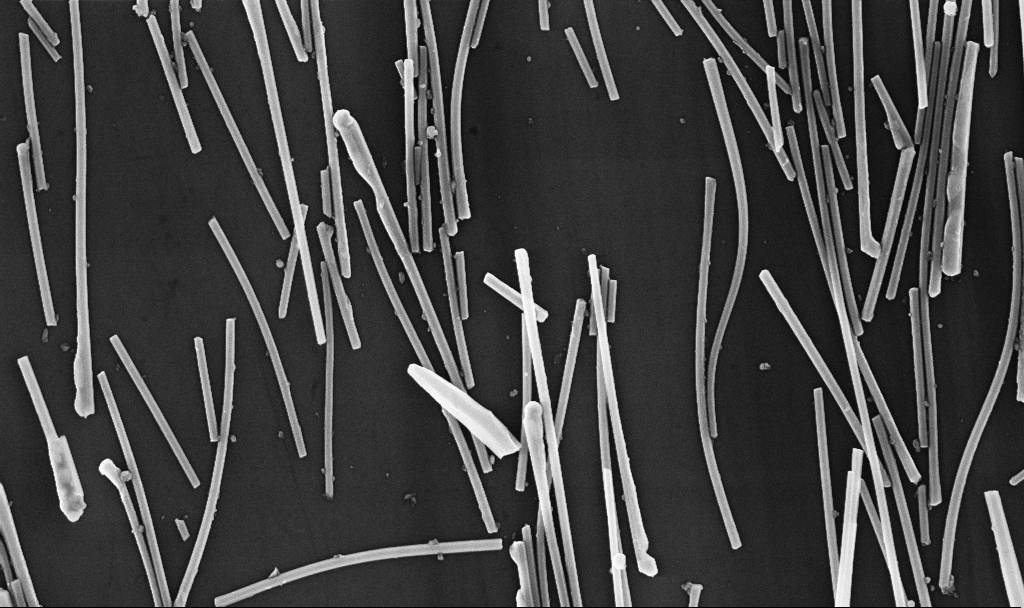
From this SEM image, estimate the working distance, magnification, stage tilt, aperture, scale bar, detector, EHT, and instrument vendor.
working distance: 6.7 mm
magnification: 31.21 K X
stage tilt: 0°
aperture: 30 µm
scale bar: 1000 nm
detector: InLens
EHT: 10 kV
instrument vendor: Zeiss